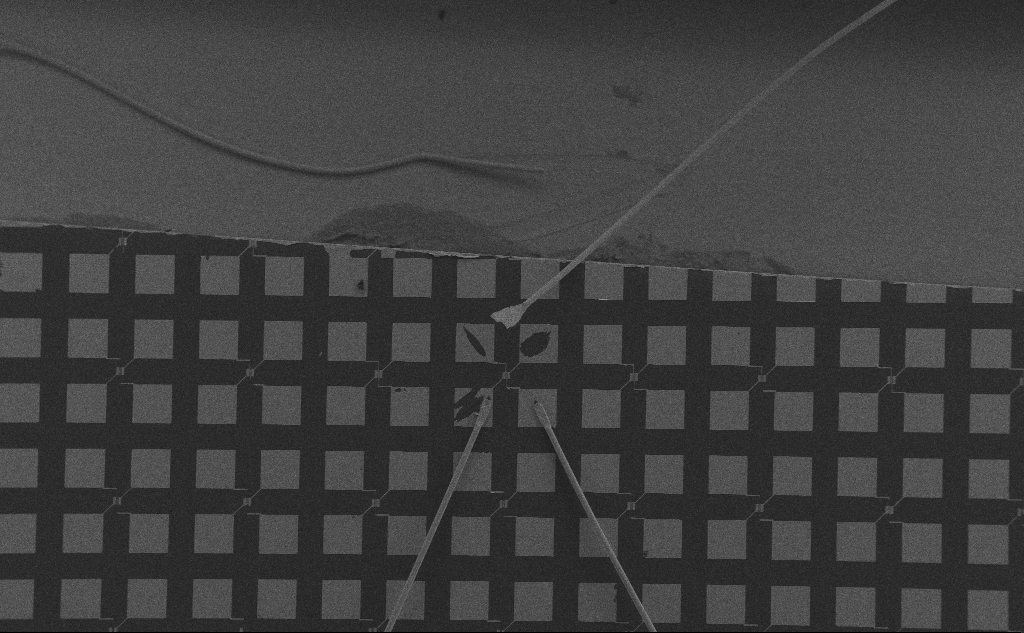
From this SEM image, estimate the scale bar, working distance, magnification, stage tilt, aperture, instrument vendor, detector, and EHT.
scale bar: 200000 nm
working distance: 6 mm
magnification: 0.096 K X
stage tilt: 0°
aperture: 30 µm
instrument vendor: Zeiss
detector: SE2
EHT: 10 kV